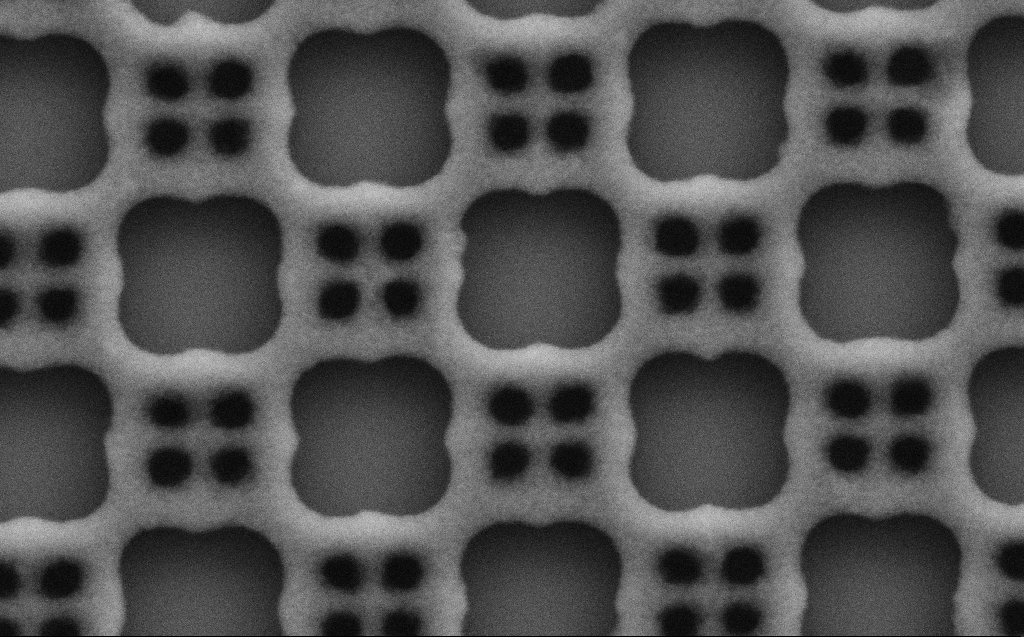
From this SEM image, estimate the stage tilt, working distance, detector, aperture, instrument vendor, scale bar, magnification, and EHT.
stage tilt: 0°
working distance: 6 mm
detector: SE2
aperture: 30 µm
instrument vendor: Zeiss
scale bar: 200 nm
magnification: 125.37 K X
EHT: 3 kV